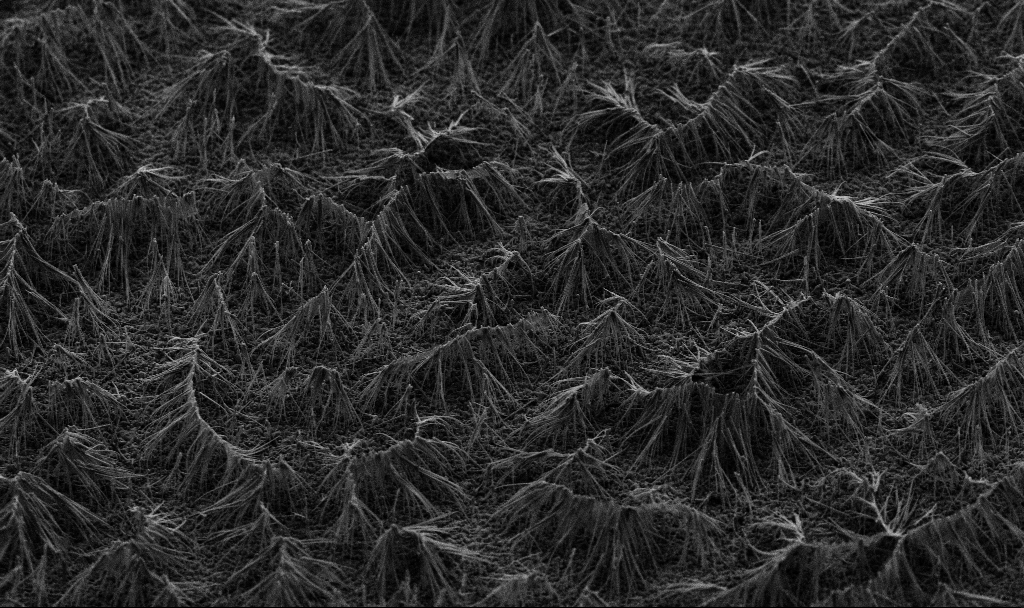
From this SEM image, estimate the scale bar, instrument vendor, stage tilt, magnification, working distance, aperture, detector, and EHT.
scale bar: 10000 nm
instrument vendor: Zeiss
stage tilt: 45°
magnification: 3.36 K X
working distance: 7.2 mm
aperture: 30 µm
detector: SE2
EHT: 5 kV